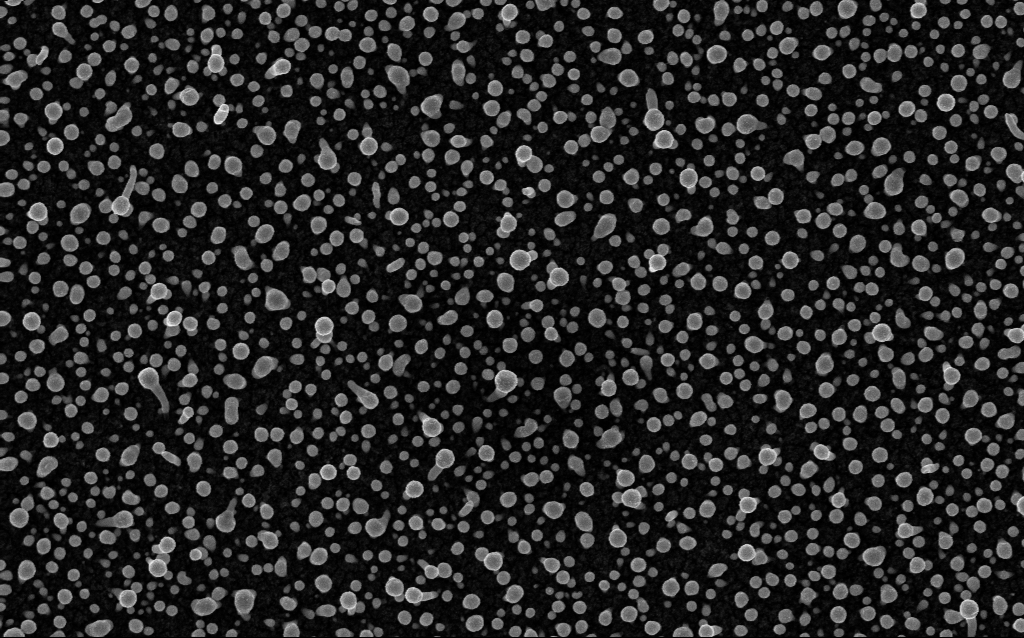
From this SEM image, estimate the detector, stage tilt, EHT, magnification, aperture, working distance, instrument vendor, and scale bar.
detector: InLens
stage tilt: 0°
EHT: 5 kV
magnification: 50 K X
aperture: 30 µm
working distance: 2.1 mm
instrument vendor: Zeiss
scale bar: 1000 nm